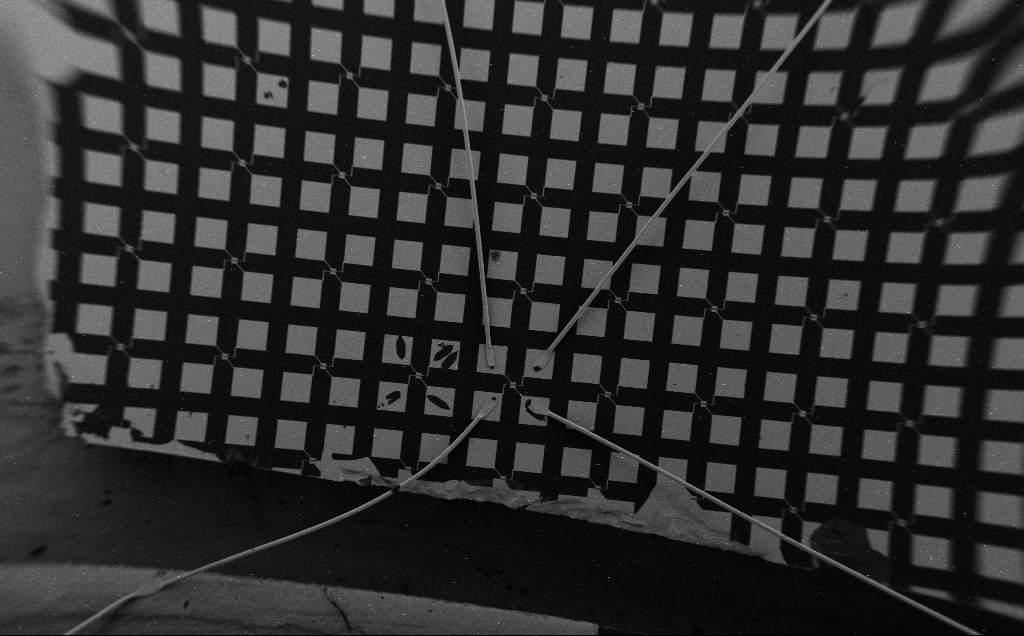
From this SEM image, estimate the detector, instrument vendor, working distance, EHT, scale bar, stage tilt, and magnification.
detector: SE2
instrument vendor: Zeiss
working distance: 8 mm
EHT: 5 kV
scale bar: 1e+06 nm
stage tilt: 0°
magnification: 0.071 K X